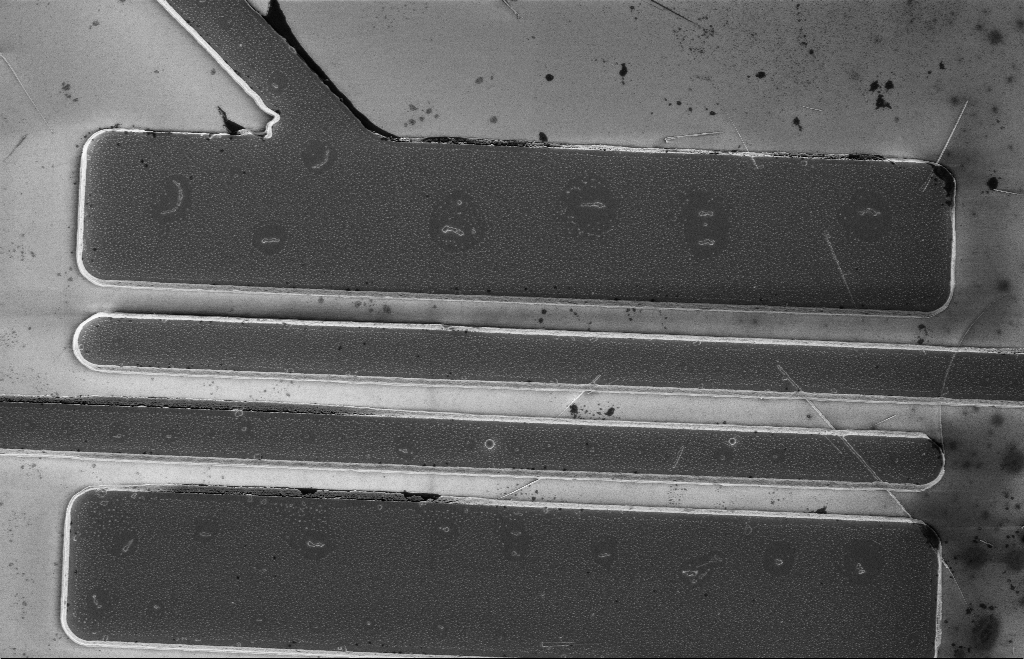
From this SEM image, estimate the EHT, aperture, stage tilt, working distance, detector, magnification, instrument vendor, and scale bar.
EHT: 5 kV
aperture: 20 µm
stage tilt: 0°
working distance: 14 mm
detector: InLens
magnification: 5.3 K X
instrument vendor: Zeiss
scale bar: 2000 nm